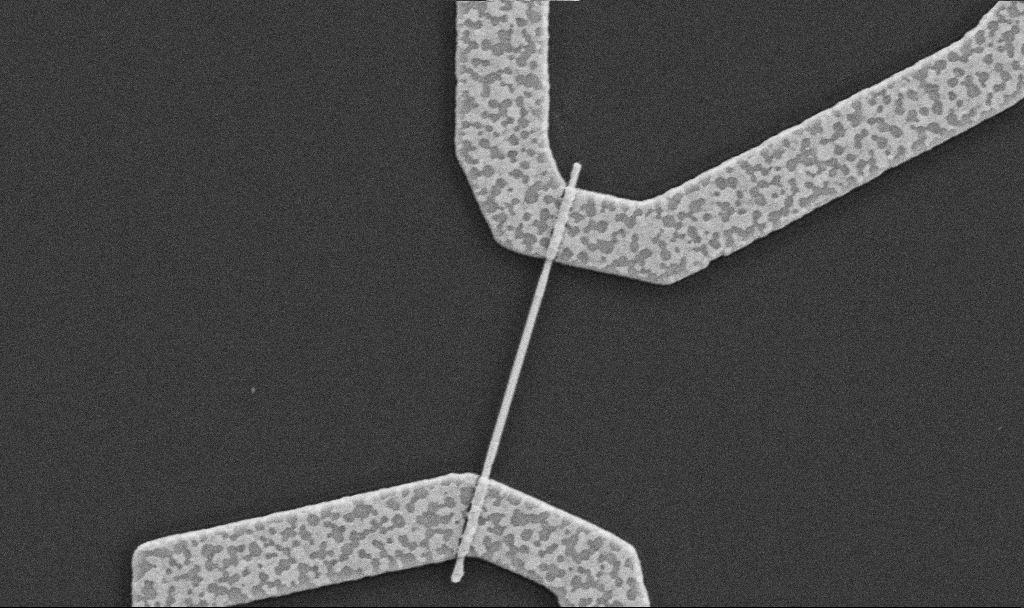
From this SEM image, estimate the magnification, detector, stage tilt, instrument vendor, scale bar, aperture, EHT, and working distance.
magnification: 30 K X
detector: SE2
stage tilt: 0°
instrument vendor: Zeiss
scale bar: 1000 nm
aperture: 30 µm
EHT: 5 kV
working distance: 8.7 mm